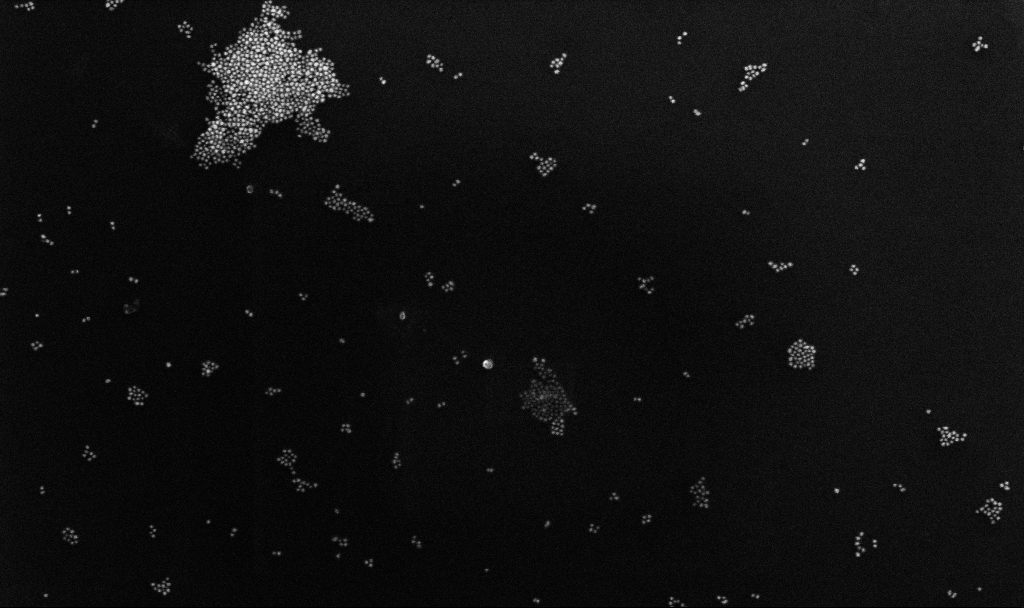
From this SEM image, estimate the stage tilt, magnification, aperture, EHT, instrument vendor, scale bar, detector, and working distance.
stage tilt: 0°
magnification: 100 K X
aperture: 30 µm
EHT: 10 kV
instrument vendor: Zeiss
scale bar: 200 nm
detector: InLens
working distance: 4.3 mm